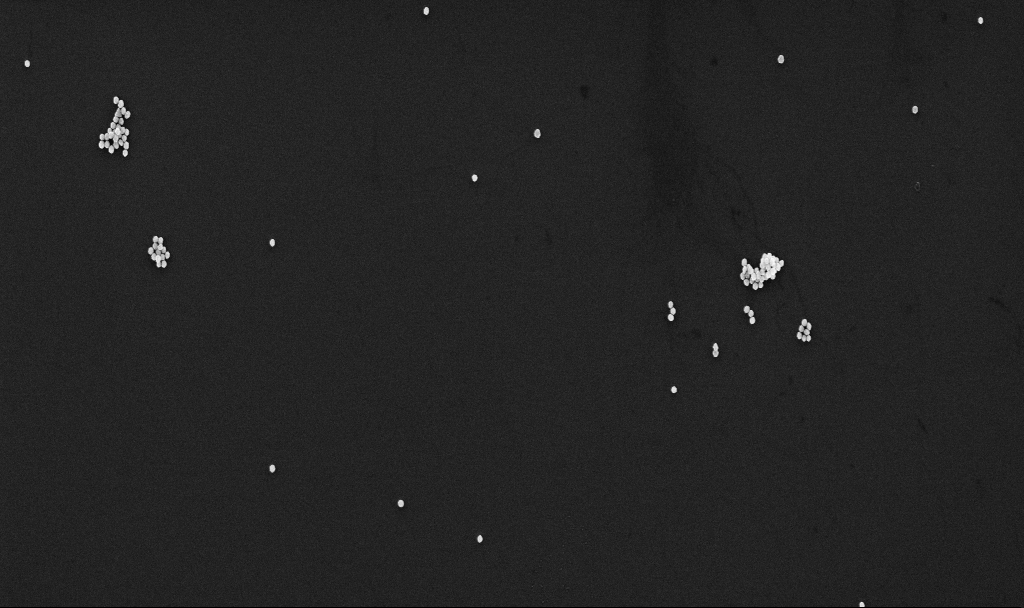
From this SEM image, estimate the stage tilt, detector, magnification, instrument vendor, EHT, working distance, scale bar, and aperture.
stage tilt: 0°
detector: InLens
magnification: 31.82 K X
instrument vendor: Zeiss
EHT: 10 kV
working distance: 4.5 mm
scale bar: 2000 nm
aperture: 30 µm